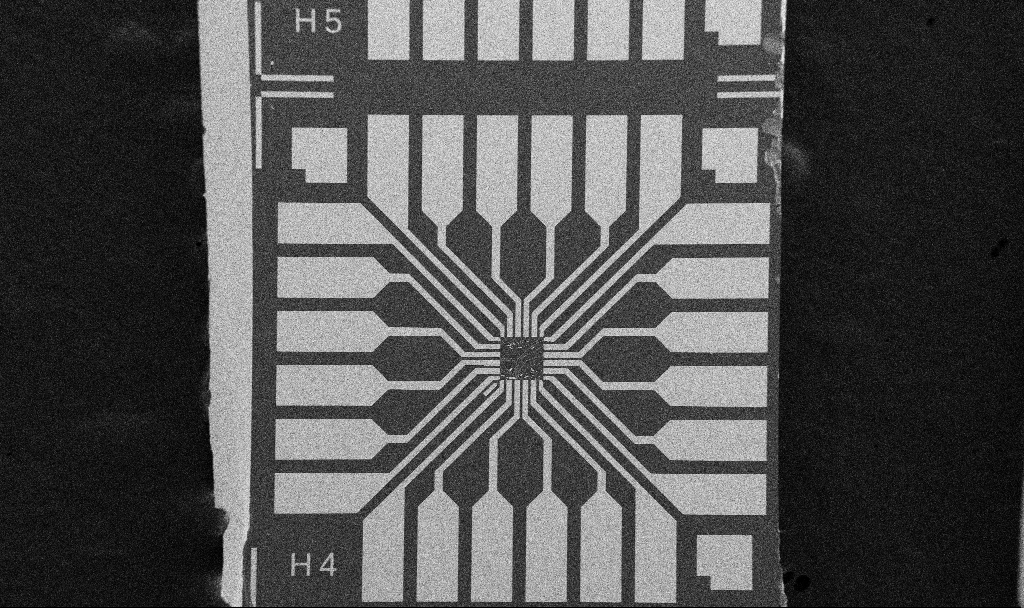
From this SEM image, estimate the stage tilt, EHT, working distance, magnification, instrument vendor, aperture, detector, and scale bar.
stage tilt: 0°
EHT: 5 kV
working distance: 10.7 mm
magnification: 0.1 K X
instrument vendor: Zeiss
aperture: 30 µm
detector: SE2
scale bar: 200000 nm